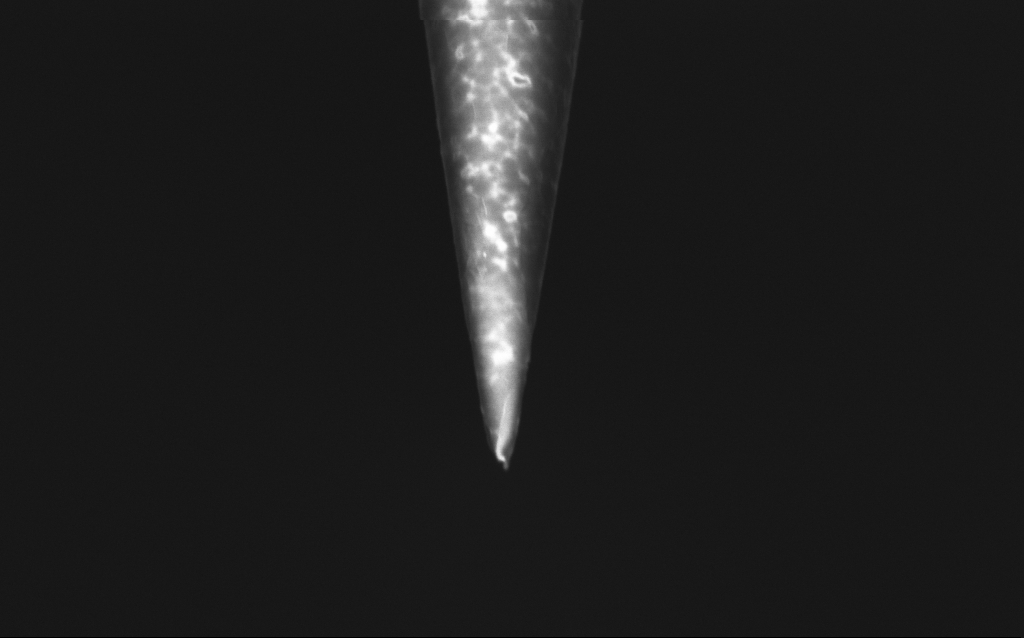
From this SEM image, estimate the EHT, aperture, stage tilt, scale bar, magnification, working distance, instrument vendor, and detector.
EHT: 1 kV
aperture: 30 µm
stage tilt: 45°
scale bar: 1000 nm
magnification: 50 K X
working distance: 6 mm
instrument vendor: Zeiss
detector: InLens